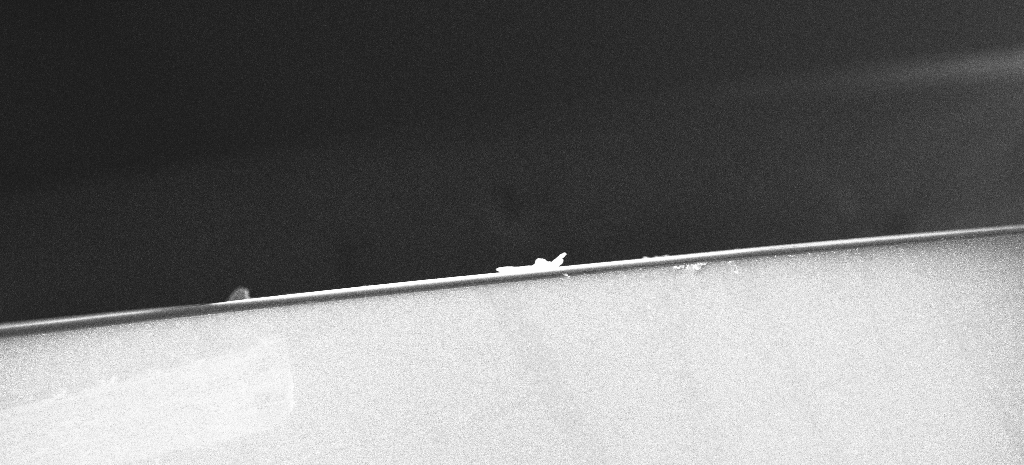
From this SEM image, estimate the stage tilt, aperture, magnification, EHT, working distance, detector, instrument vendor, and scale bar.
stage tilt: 0°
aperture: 30 µm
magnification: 0.2 K X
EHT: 20 kV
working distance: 1.5 mm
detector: InLens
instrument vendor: Zeiss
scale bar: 100000 nm